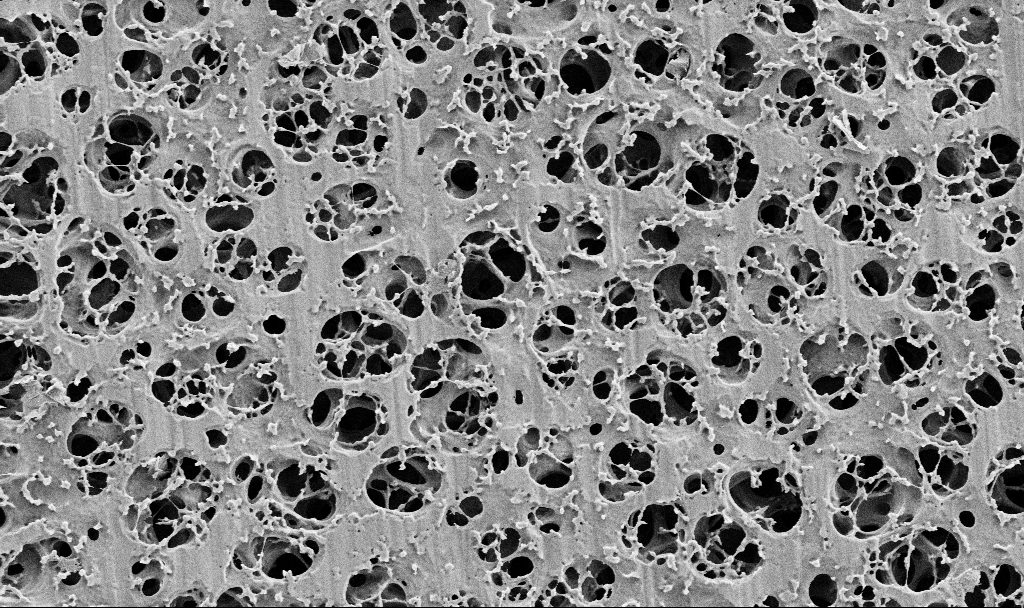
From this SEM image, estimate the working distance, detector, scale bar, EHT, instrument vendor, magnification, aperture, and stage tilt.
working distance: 3.7 mm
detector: SE2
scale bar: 2000 nm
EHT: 2 kV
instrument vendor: Zeiss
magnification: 10 K X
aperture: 30 µm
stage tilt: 0°